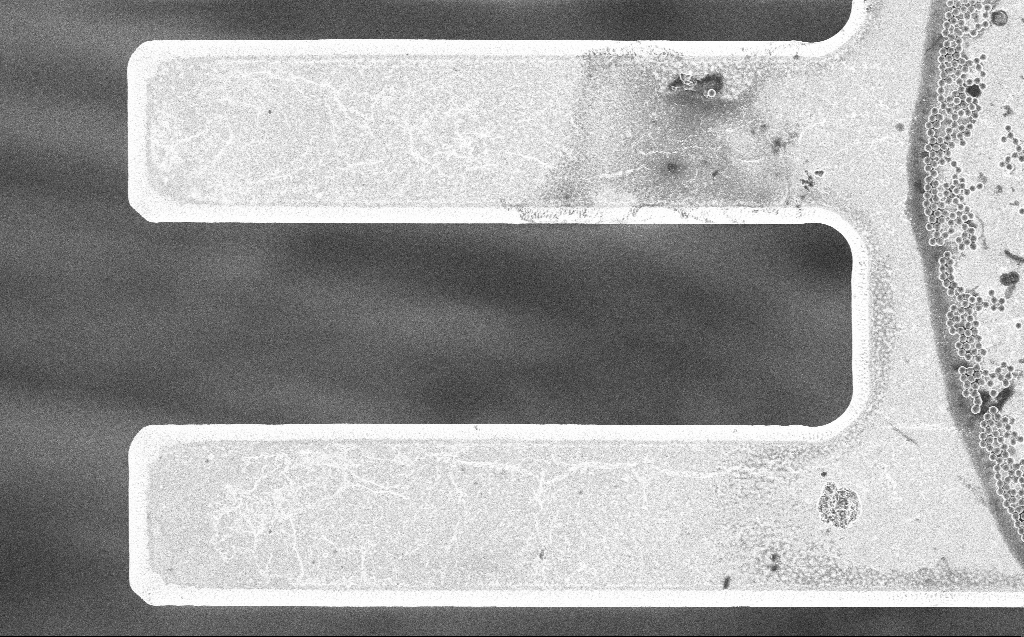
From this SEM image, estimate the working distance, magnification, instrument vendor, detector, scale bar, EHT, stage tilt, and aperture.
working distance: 7 mm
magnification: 3.54 K X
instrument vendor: Zeiss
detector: InLens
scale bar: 20000 nm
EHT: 3 kV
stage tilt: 0°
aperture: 30 µm